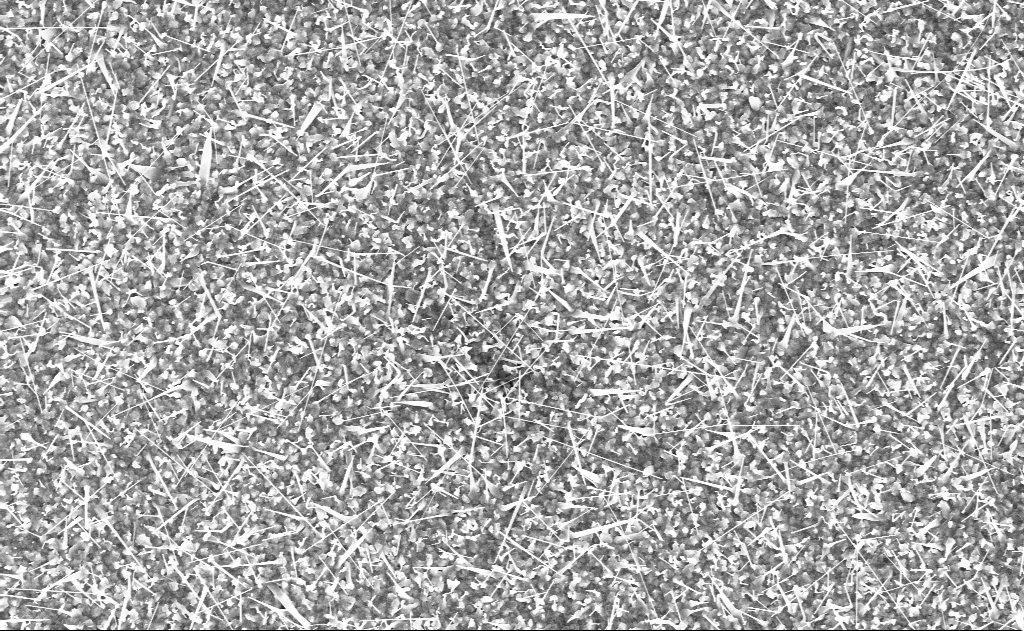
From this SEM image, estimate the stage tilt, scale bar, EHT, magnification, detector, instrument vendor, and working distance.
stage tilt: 0°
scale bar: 2000 nm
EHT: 10 kV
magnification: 10 K X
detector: InLens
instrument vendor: Zeiss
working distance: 12 mm